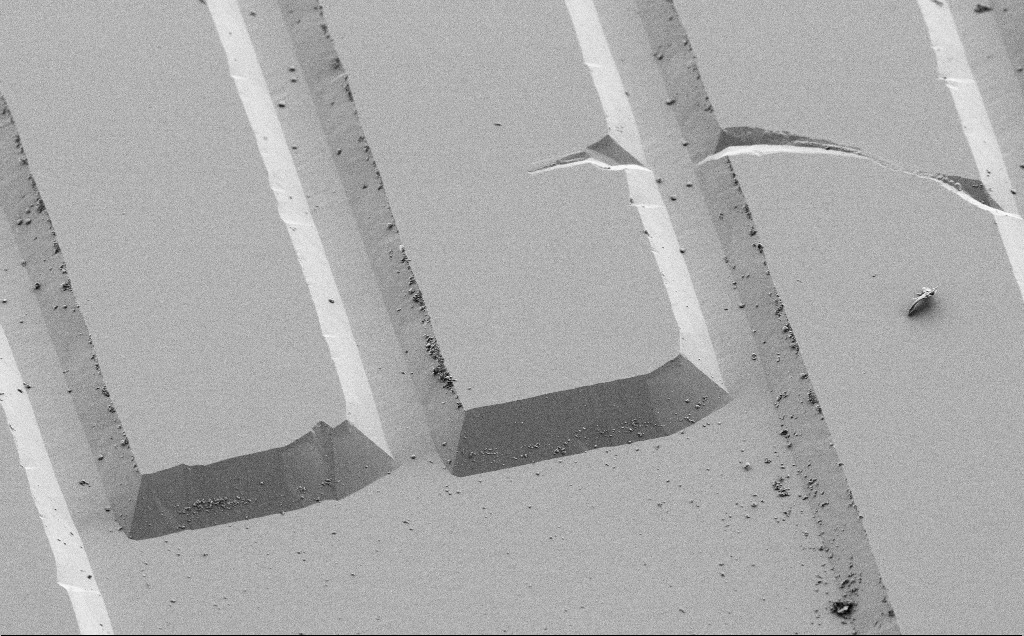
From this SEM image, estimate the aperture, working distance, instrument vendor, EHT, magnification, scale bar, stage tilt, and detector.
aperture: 30 µm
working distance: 9 mm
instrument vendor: Zeiss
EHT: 3 kV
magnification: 1.17 K X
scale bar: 20000 nm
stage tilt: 45°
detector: SE2